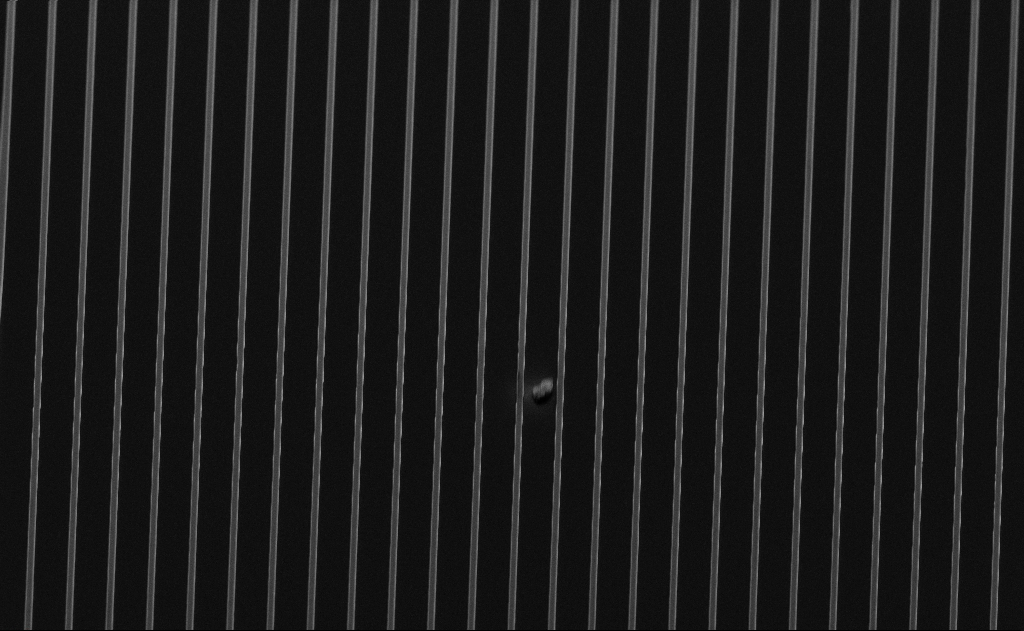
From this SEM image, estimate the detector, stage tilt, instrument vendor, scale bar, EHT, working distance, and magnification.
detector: SE2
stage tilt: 50°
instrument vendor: Zeiss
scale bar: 10000 nm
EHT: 5 kV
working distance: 12 mm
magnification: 1.85 K X